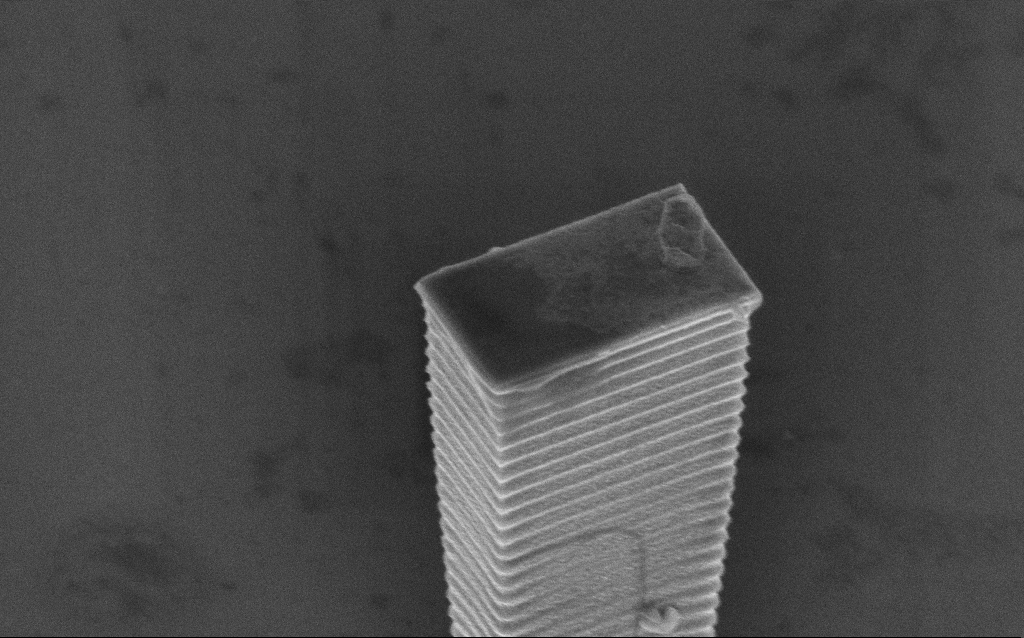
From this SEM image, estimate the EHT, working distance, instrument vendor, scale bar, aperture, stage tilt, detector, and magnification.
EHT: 5 kV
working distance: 12 mm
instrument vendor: Zeiss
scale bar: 2000 nm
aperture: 30 µm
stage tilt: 45°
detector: InLens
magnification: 22.54 K X